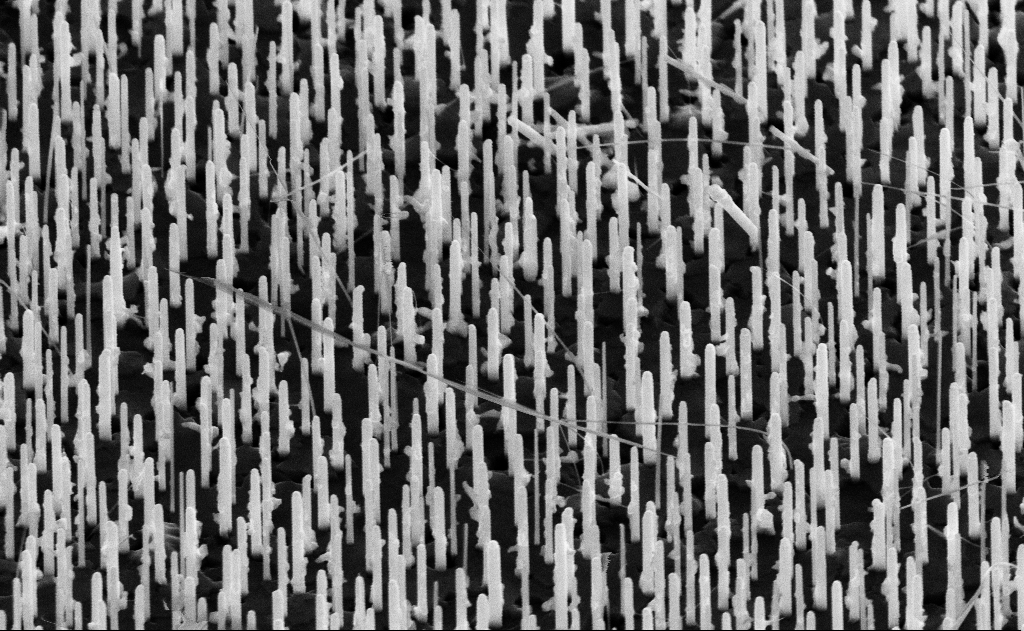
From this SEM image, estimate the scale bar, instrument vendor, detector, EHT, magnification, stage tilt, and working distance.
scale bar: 1000 nm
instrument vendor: Zeiss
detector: SE2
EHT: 10 kV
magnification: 40 K X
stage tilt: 45°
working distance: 14 mm